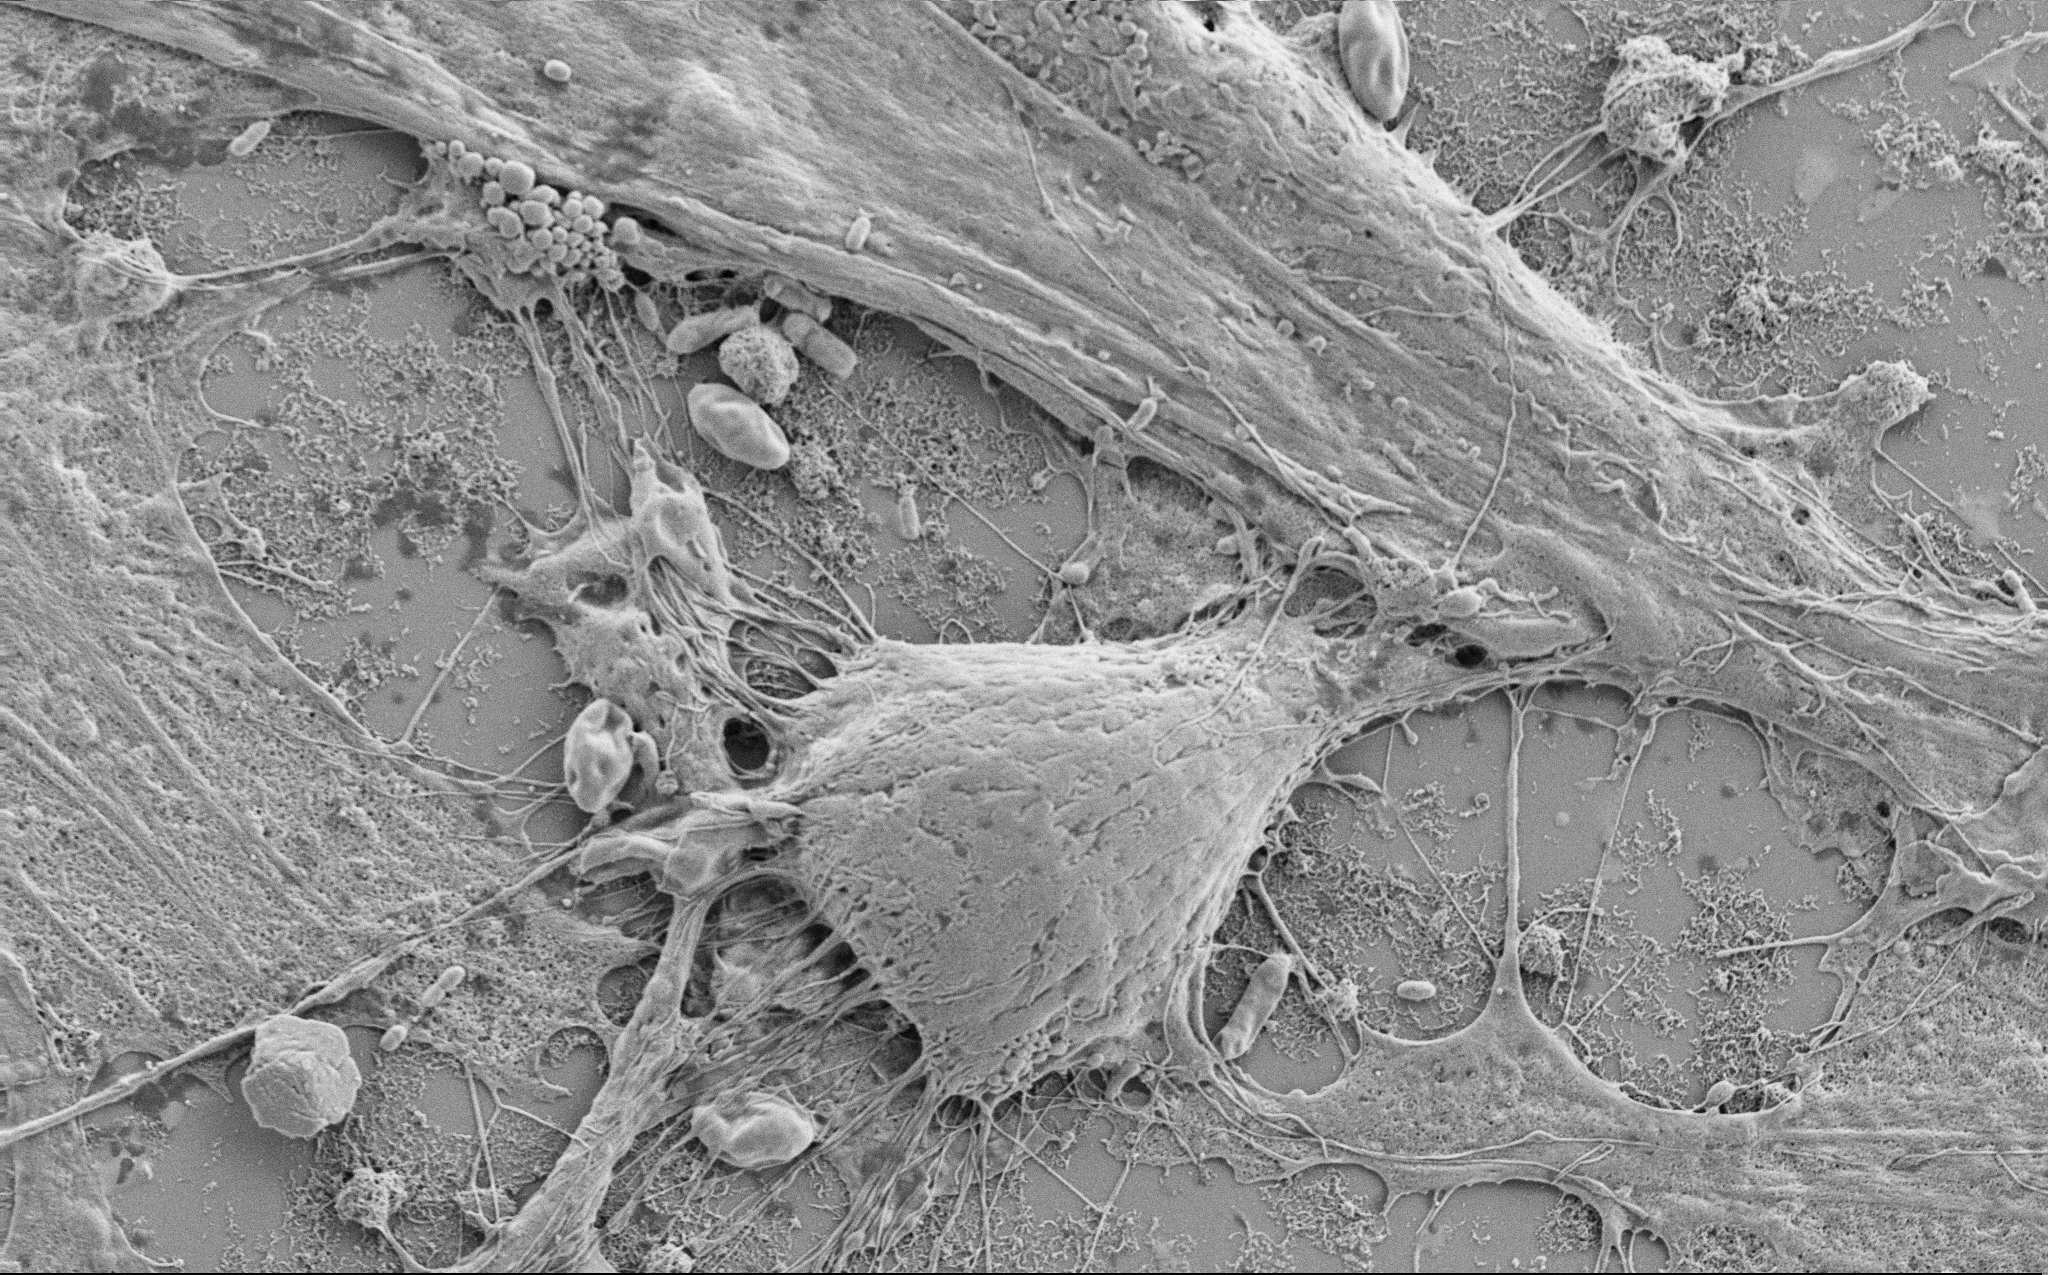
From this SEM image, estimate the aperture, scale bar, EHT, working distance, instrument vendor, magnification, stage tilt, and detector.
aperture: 30 µm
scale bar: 10000 nm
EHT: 2 kV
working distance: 6.9 mm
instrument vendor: Zeiss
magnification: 5 K X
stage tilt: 0°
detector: SE2